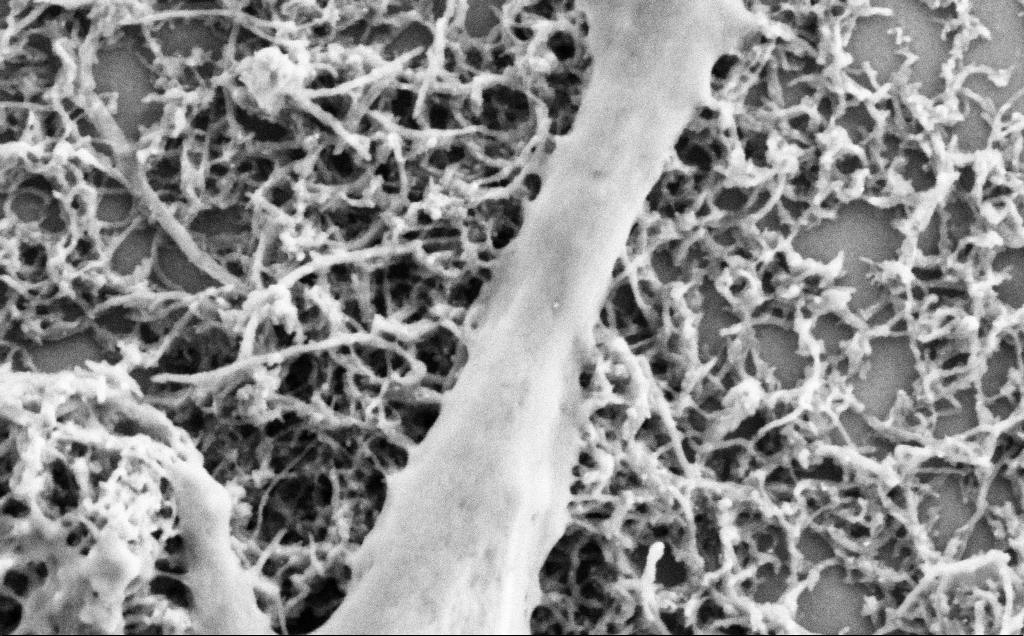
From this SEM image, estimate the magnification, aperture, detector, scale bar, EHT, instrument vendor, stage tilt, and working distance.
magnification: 75 K X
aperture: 30 µm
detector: SE2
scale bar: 200 nm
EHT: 2 kV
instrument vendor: Zeiss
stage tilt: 0°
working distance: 7.1 mm